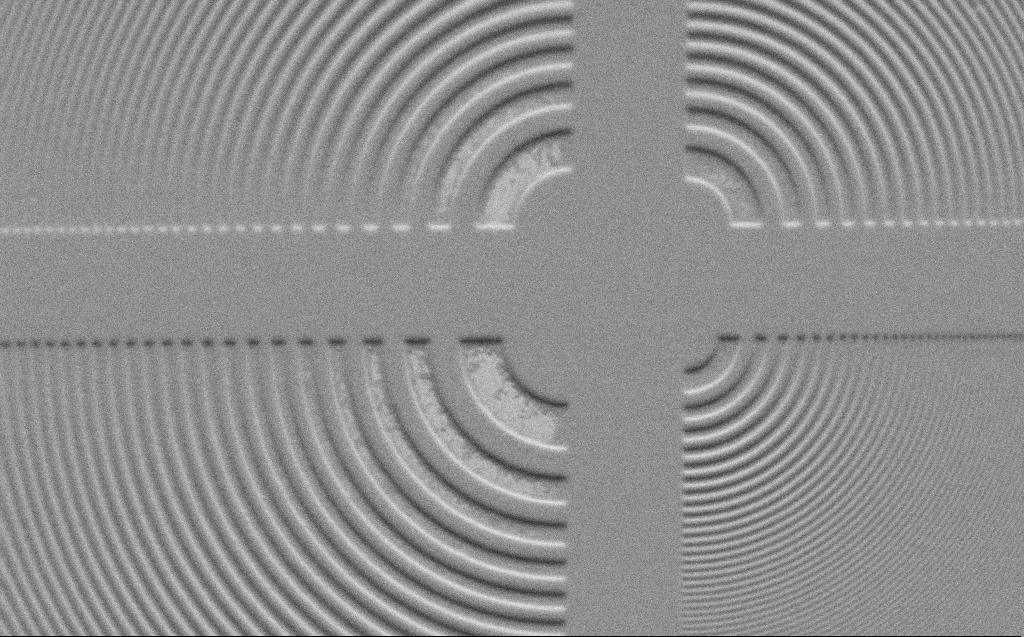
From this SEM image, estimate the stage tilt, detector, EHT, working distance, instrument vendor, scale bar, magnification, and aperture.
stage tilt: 45°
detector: SE2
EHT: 1 kV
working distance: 5 mm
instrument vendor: Zeiss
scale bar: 10000 nm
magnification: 4.25 K X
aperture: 30 µm